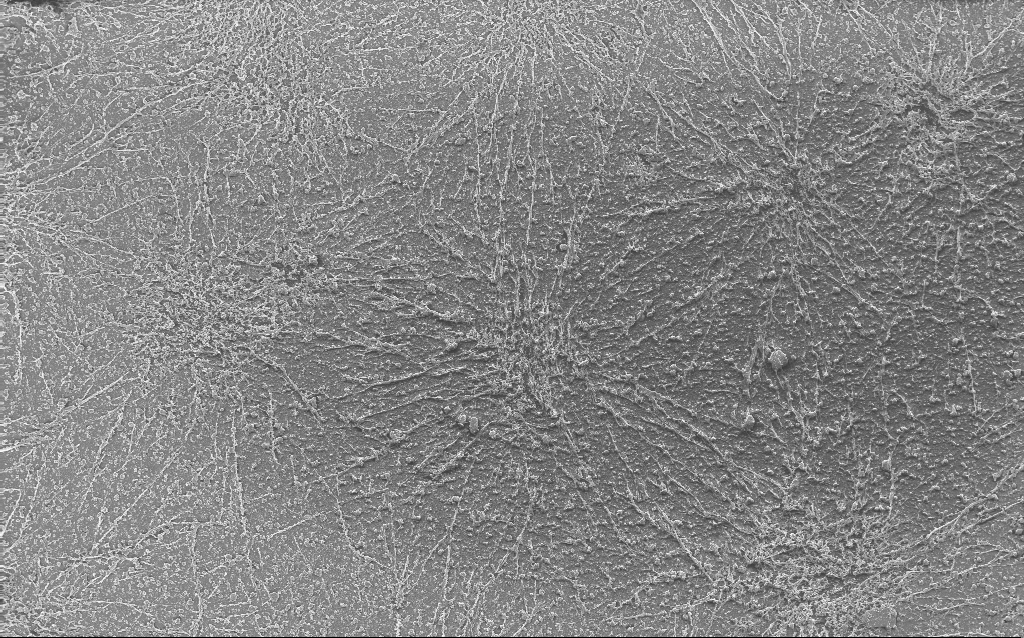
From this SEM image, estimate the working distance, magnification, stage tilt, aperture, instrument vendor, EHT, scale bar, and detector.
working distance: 2.7 mm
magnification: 0.235 K X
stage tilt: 0°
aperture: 30 µm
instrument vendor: Zeiss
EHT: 10 kV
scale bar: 100000 nm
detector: InLens